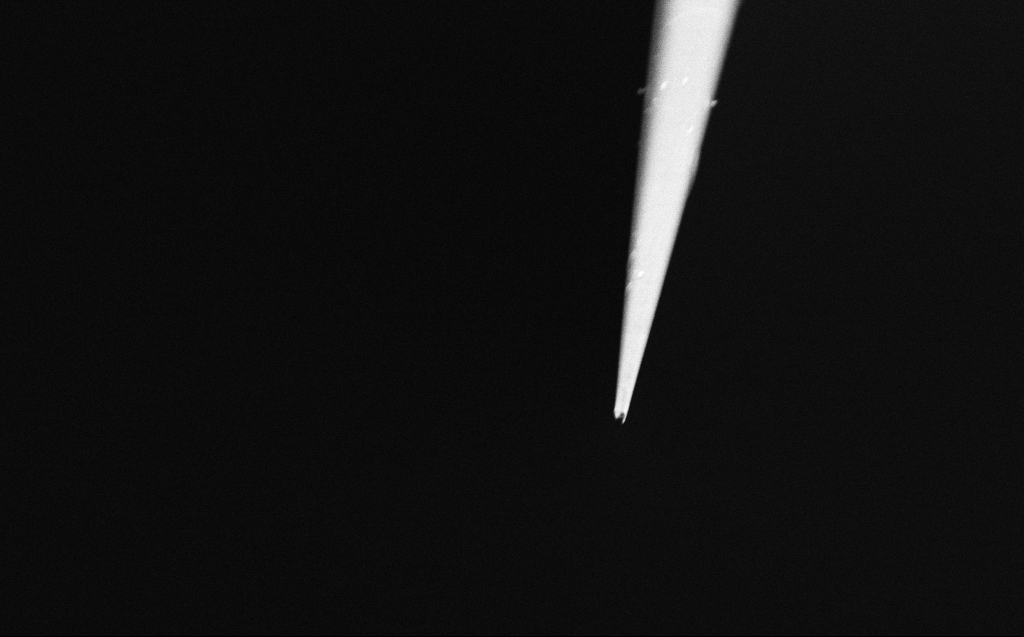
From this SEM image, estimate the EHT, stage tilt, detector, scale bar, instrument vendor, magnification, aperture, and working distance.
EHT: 2 kV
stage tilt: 45°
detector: InLens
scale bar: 2000 nm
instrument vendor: Zeiss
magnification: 25 K X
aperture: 30 µm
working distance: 4 mm